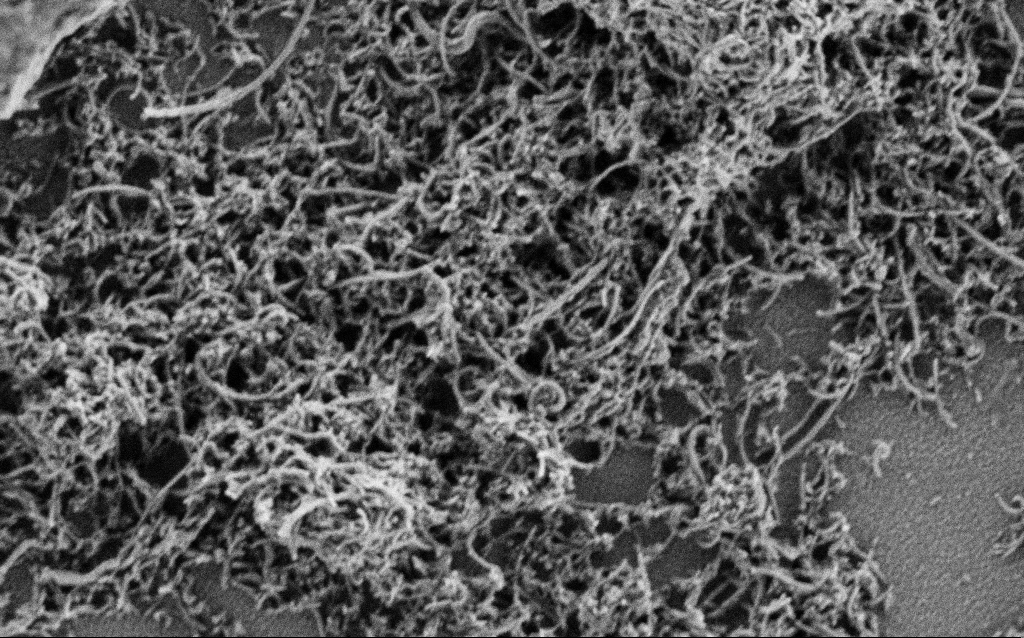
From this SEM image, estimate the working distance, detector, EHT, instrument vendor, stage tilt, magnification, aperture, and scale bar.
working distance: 4 mm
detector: SE2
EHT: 1 kV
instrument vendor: Zeiss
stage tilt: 0°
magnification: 50 K X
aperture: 30 µm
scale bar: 1000 nm